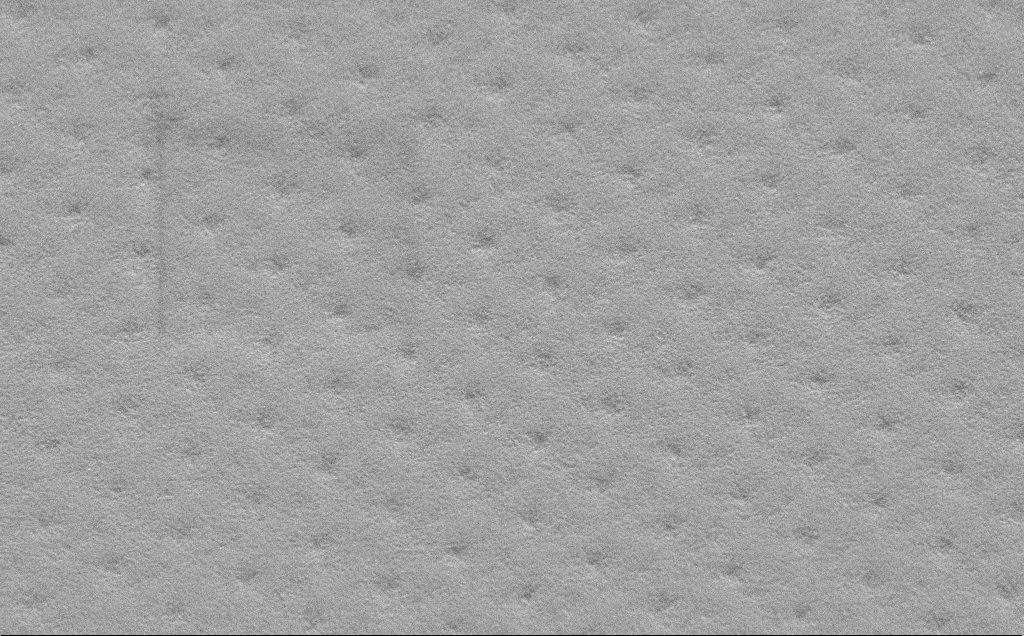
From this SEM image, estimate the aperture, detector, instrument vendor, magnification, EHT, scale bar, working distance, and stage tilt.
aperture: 30 µm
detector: SE2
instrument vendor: Zeiss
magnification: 14.52 K X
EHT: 5 kV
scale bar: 2000 nm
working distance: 9 mm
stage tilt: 45°